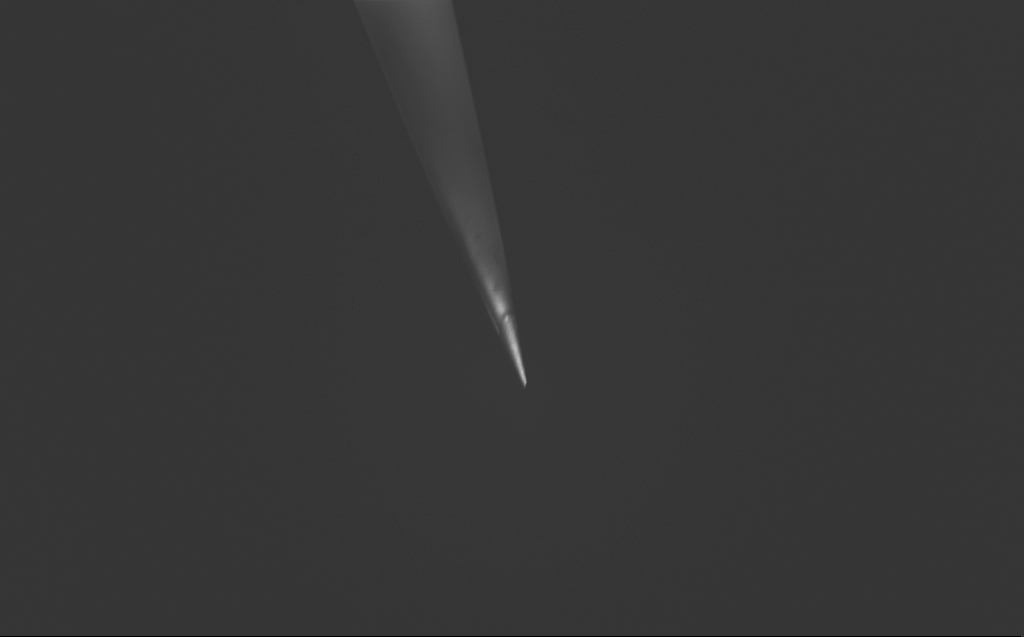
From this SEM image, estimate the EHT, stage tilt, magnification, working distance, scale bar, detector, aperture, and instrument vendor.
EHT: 0.8 kV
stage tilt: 39.3°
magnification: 10 K X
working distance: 5 mm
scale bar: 2000 nm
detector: InLens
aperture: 30 µm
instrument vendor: Zeiss